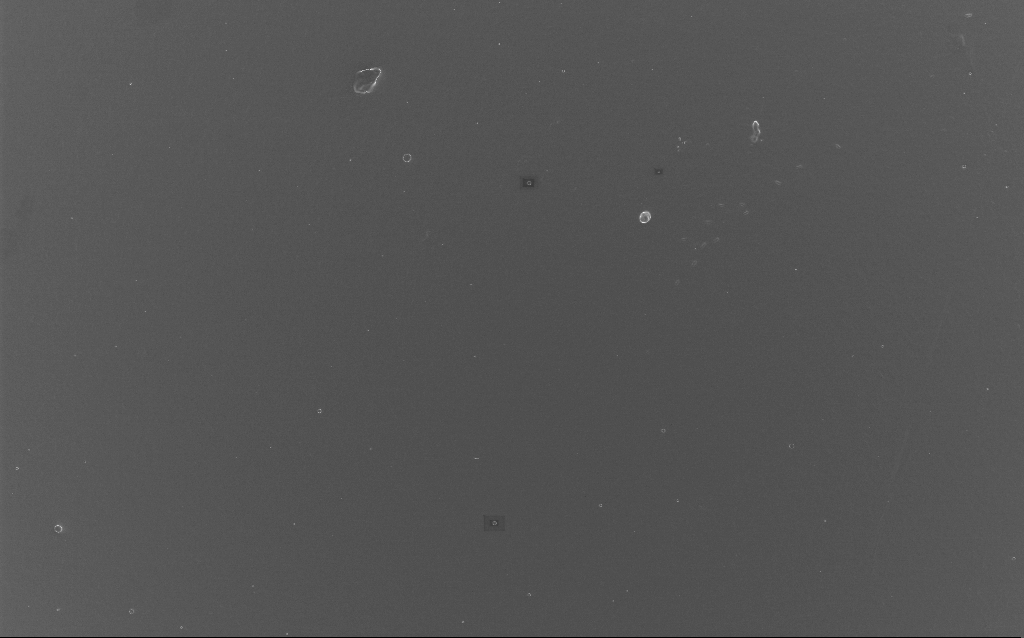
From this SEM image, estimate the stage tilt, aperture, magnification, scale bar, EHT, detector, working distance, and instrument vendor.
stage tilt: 0°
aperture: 30 µm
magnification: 1.15 K X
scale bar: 20000 nm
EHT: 10 kV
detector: InLens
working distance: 2 mm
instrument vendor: Zeiss